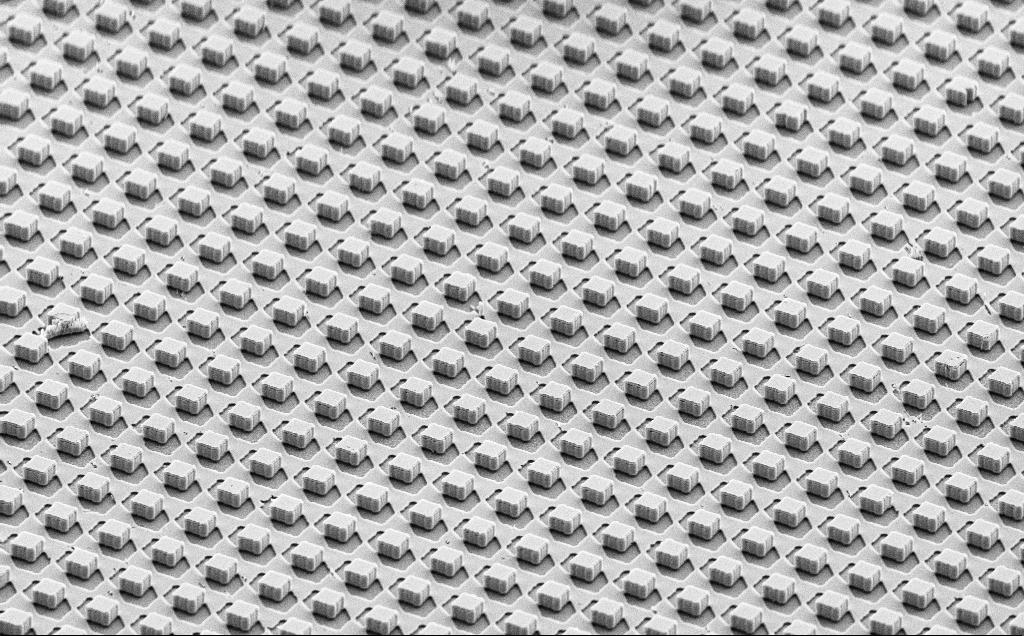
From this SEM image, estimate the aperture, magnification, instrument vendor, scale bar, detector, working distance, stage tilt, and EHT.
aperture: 30 µm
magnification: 1.15 K X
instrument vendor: Zeiss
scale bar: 20000 nm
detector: SE2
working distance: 10 mm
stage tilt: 55.6°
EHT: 10 kV